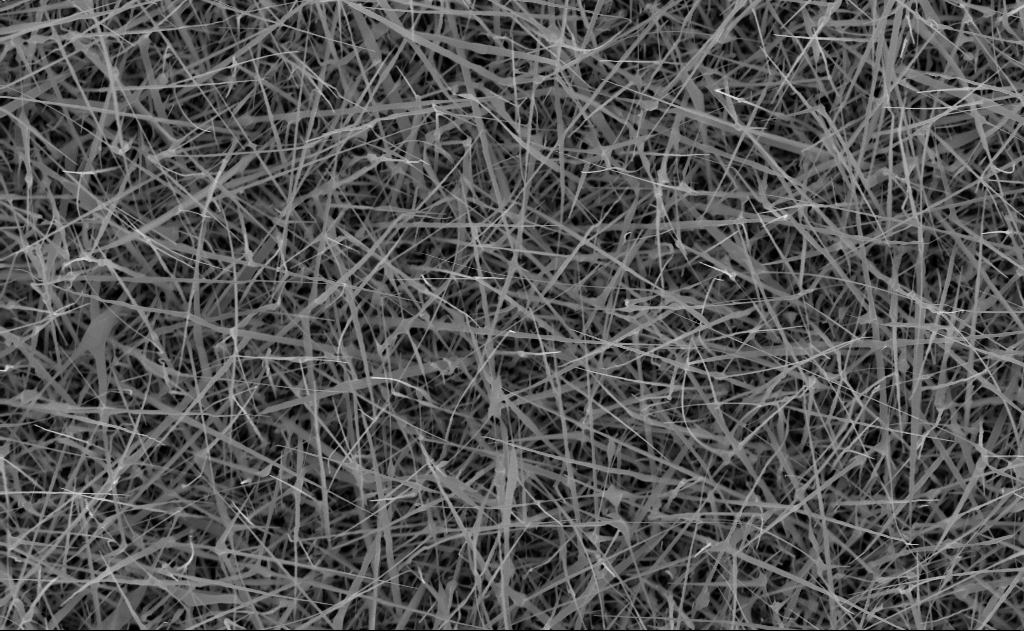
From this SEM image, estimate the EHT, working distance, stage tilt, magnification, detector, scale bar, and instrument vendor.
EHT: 10 kV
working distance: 10 mm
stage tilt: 0°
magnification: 20 K X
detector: InLens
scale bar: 2000 nm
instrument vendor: Zeiss